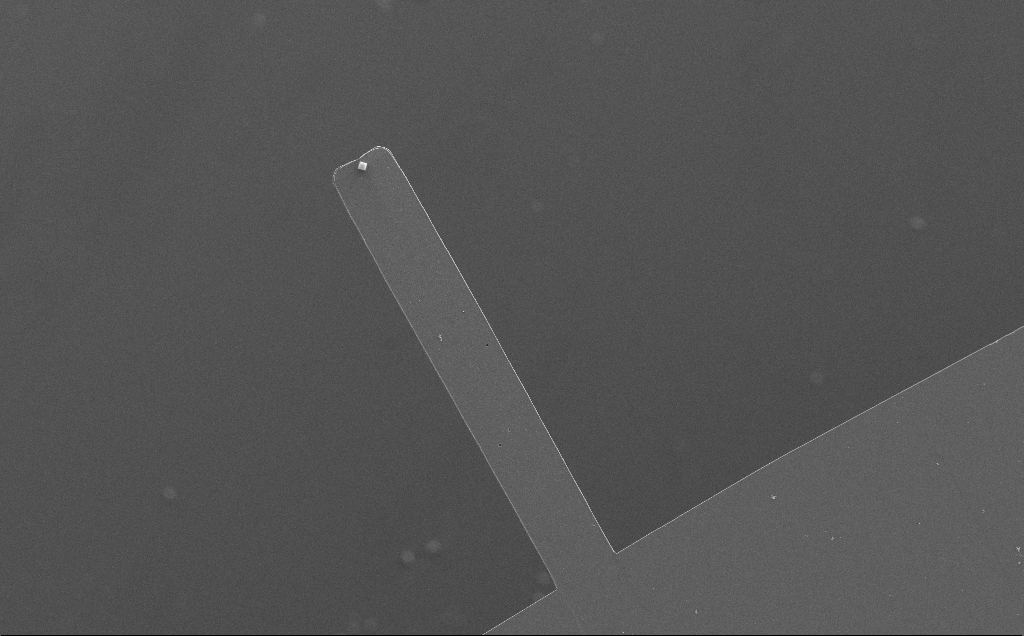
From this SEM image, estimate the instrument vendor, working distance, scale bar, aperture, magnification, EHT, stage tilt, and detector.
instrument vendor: Zeiss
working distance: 10 mm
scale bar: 100000 nm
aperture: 30 µm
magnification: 0.666 K X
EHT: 5 kV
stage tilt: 0°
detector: SE2